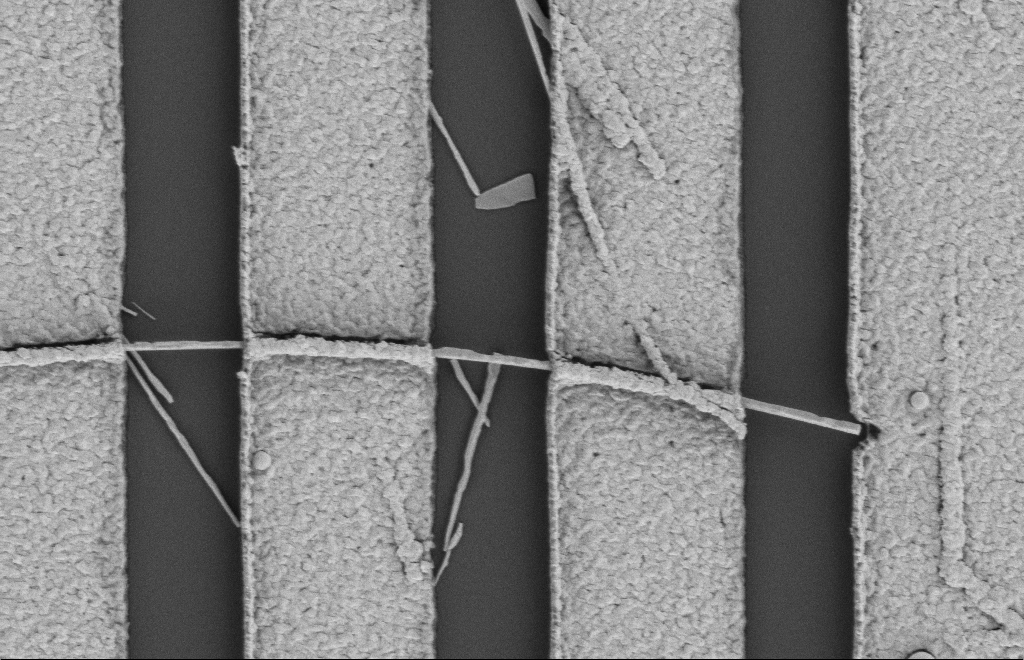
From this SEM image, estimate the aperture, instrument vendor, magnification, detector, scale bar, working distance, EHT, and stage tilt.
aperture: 20 µm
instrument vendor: Zeiss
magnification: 27.74 K X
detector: SE2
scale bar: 1000 nm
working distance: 12 mm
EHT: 2 kV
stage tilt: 0°